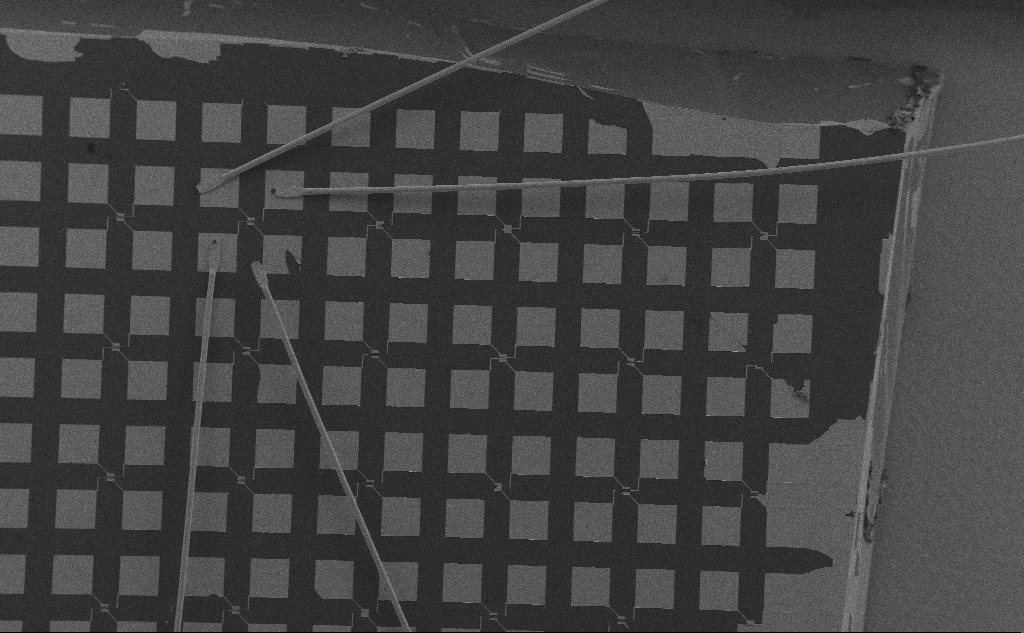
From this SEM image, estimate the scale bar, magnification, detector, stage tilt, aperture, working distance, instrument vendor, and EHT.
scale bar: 200000 nm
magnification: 0.096 K X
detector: SE2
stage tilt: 0°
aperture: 30 µm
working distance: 6 mm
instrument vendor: Zeiss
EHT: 10 kV